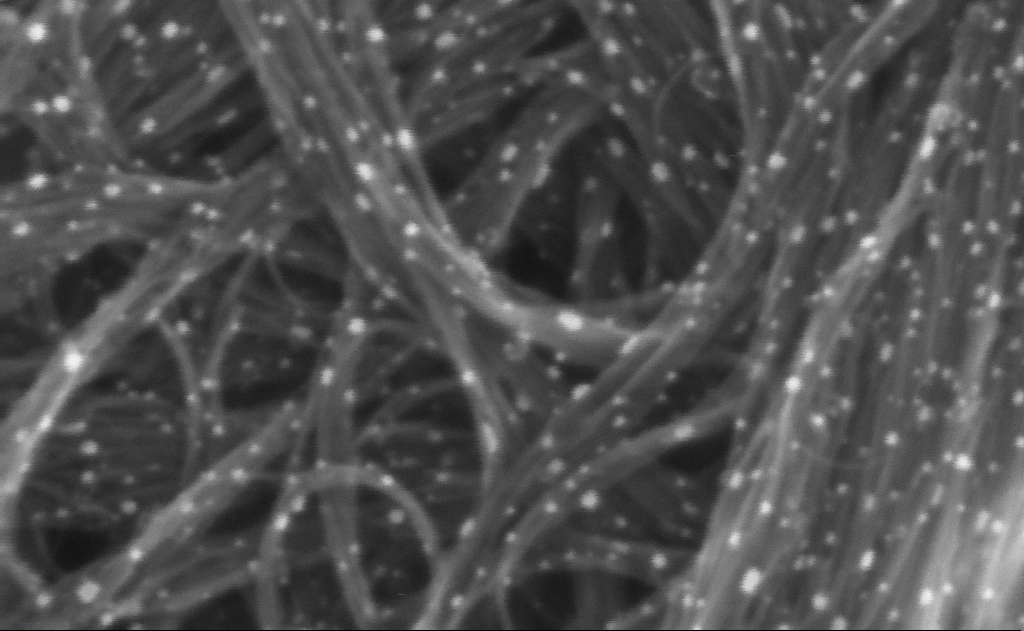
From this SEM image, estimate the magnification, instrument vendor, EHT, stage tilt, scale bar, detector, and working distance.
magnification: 988.46 K X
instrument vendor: Zeiss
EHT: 10 kV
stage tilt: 0°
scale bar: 20 nm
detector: InLens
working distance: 3 mm